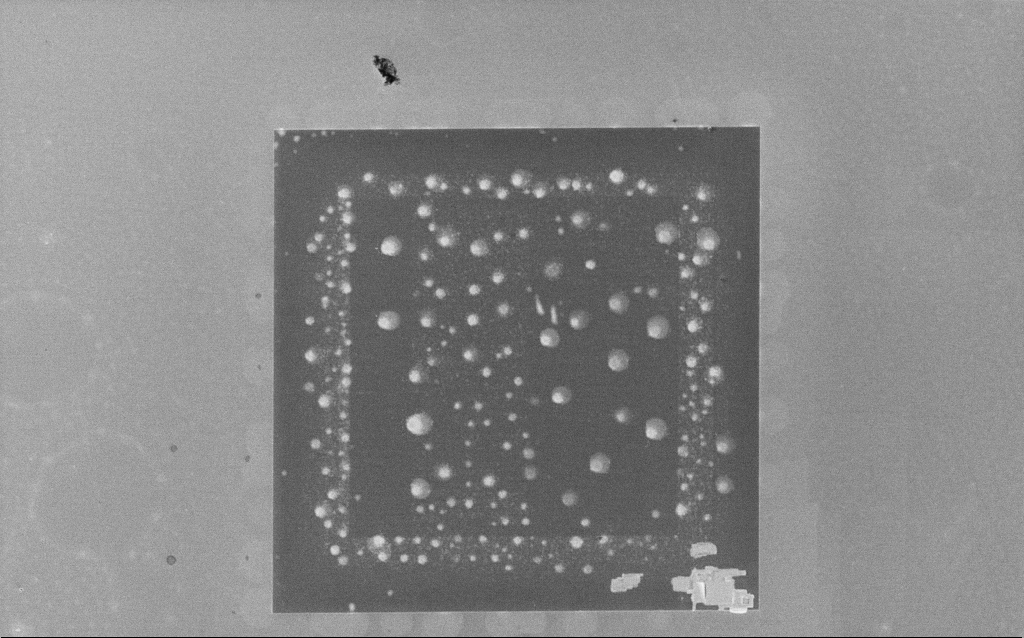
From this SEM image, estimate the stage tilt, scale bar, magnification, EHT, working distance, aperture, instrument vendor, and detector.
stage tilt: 0°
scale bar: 100000 nm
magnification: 0.599 K X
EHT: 3 kV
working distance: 5.1 mm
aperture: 30 µm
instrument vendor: Zeiss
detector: InLens